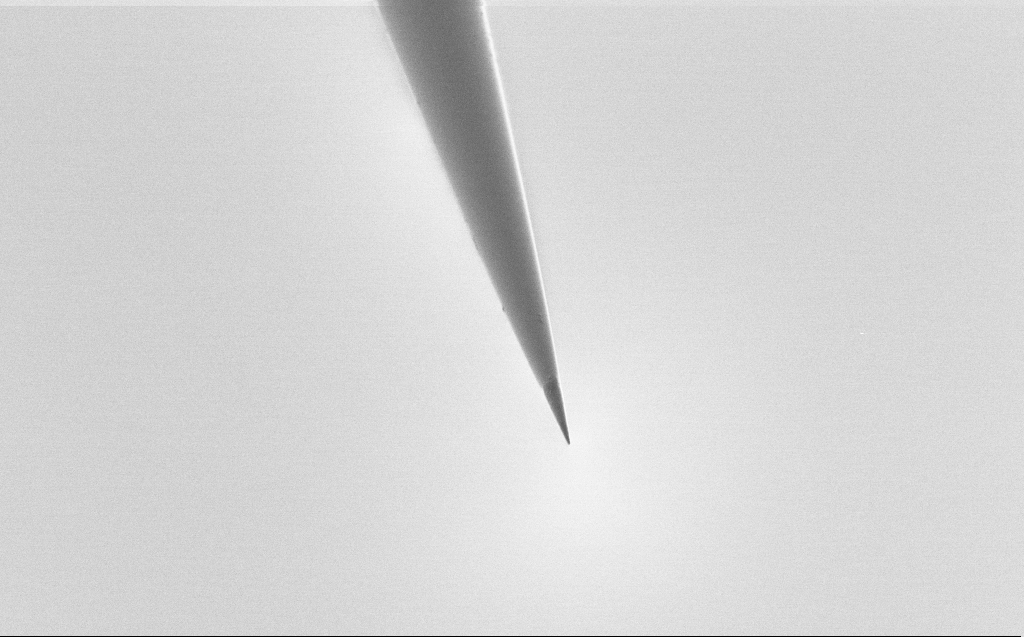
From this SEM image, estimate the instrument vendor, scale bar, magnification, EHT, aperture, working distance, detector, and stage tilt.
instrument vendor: Zeiss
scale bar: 2000 nm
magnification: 10 K X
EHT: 0.8 kV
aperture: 30 µm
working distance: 5 mm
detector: SE2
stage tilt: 39.3°